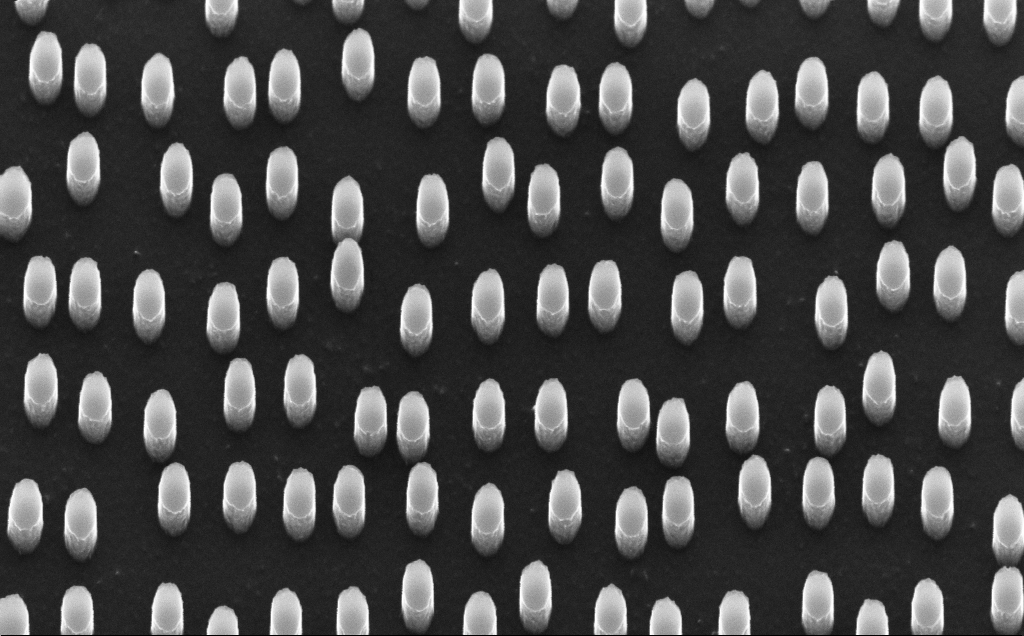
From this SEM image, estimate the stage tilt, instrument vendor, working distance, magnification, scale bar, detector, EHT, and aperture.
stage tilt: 30°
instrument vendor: Zeiss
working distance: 5 mm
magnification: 60.27 K X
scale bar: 1000 nm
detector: InLens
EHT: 10 kV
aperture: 30 µm